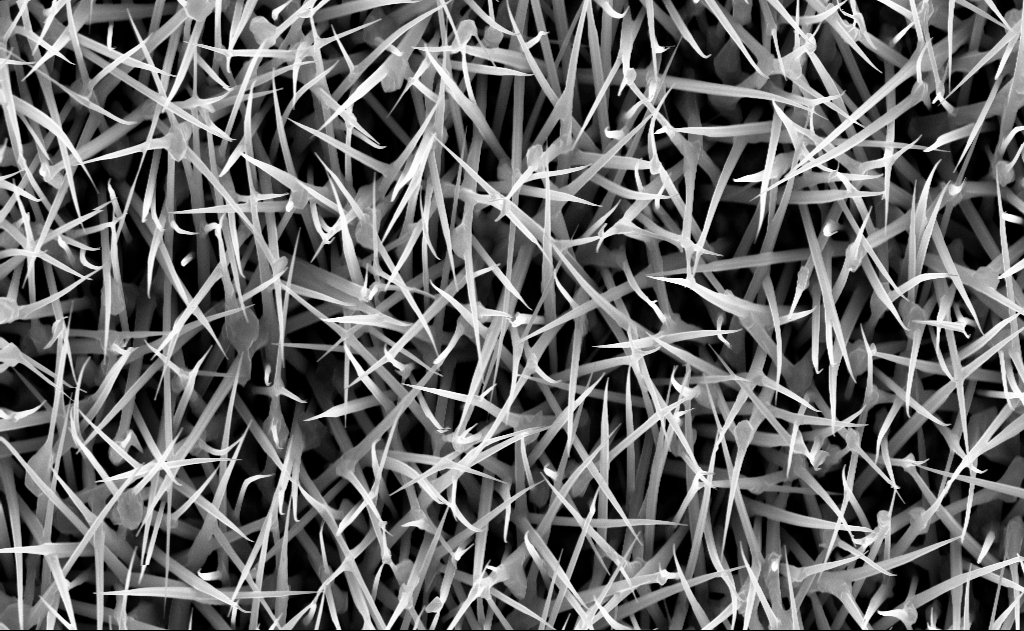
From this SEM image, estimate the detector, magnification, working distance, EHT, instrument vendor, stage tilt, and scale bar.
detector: InLens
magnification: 20 K X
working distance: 7 mm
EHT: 10 kV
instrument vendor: Zeiss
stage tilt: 0°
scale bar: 2000 nm